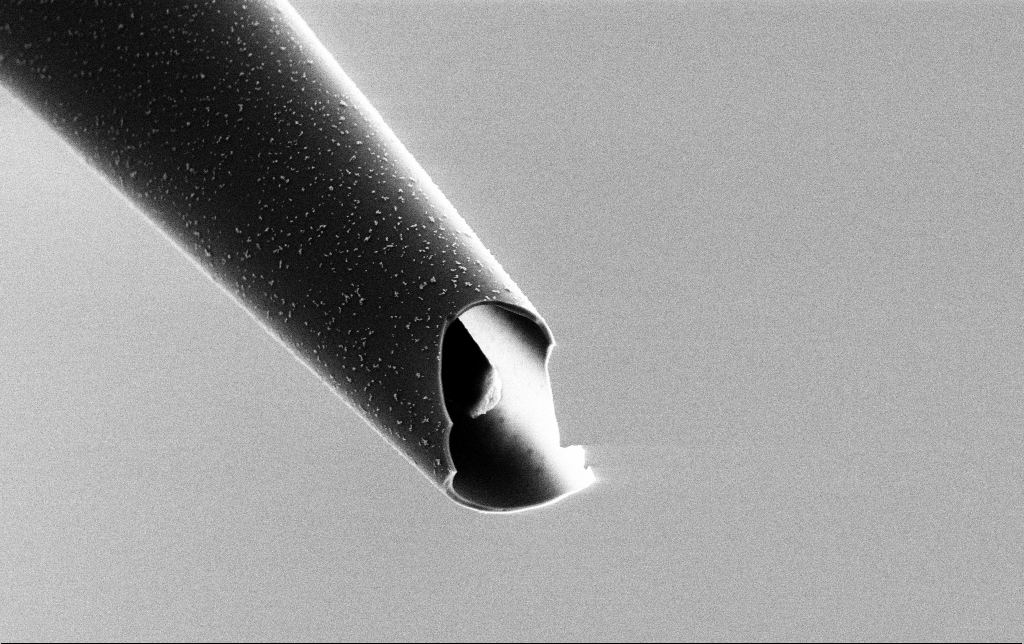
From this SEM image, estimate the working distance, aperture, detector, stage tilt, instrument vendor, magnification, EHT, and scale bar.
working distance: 7.7 mm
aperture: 30 µm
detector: SE2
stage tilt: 45°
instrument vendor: Zeiss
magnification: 15 K X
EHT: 3 kV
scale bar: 2000 nm